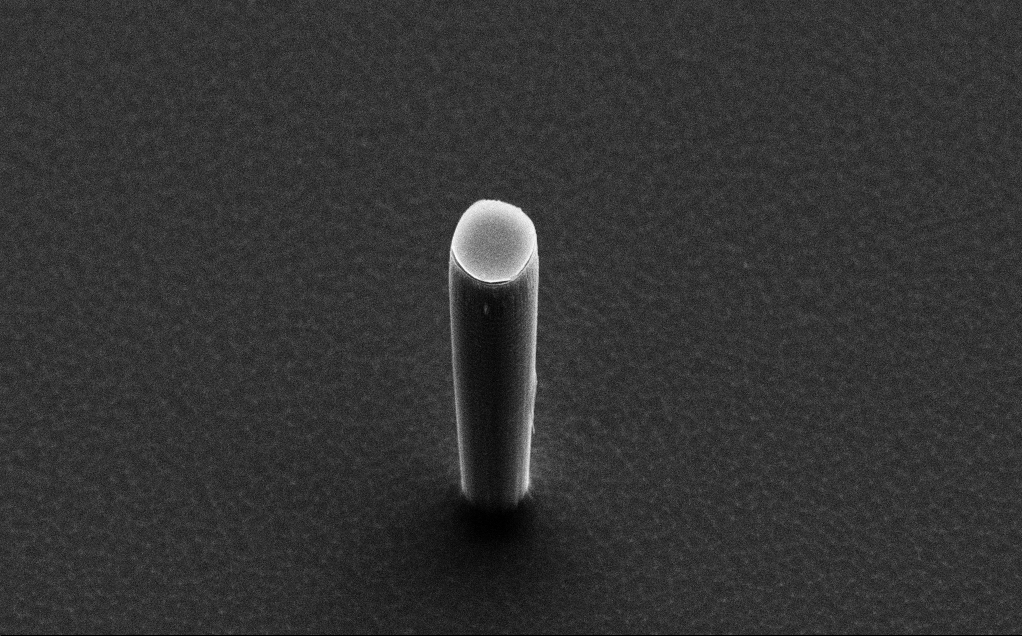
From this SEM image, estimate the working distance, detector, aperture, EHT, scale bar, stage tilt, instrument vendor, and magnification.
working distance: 10 mm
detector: SE2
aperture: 30 µm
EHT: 15 kV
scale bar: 10000 nm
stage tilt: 50°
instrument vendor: Zeiss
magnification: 4.74 K X